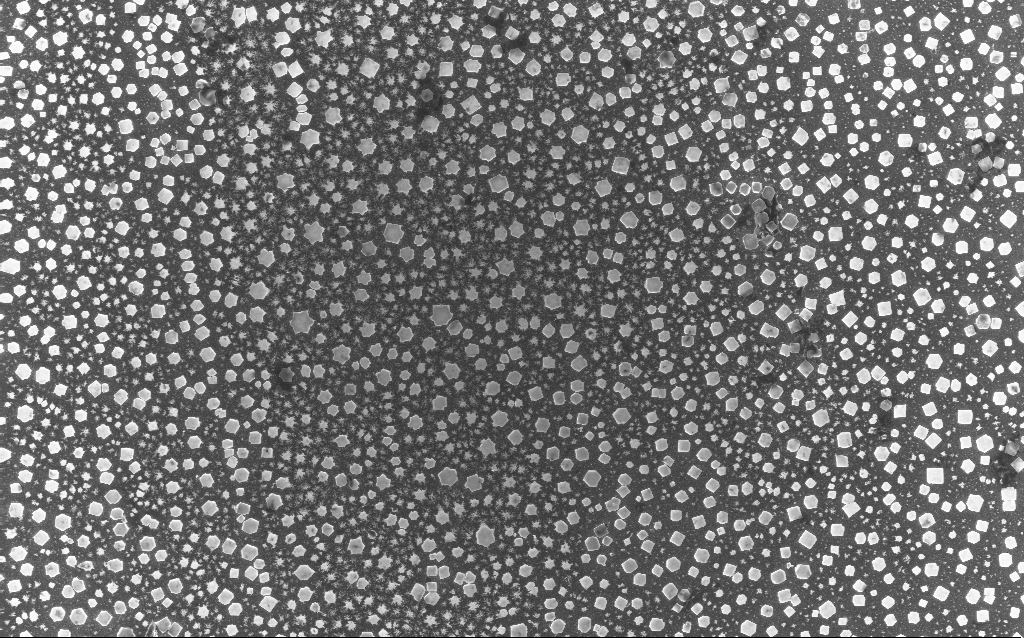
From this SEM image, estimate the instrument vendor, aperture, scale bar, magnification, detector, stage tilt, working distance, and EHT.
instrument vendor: Zeiss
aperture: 30 µm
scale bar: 20000 nm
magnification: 0.77 K X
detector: InLens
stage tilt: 0°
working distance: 4.5 mm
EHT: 10 kV